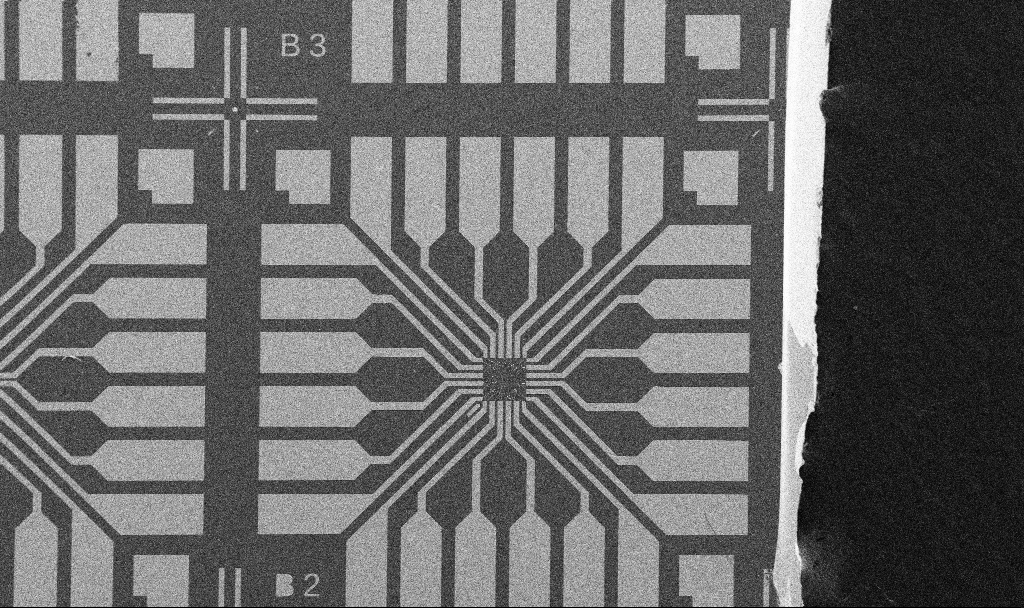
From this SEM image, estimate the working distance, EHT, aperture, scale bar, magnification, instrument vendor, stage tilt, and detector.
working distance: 10.7 mm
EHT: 10 kV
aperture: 30 µm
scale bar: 200000 nm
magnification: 0.1 K X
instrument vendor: Zeiss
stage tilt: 0°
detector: SE2